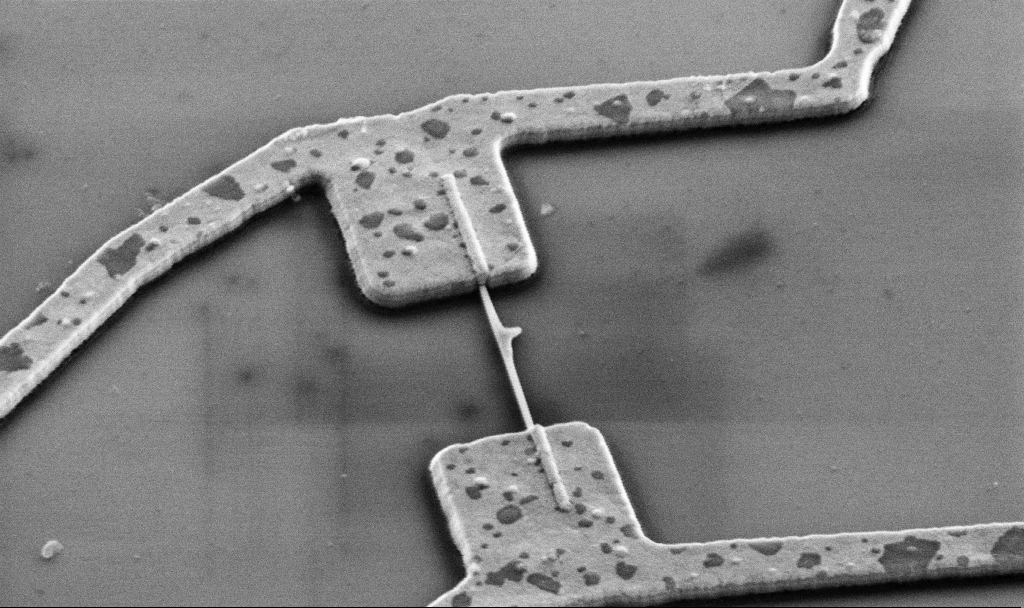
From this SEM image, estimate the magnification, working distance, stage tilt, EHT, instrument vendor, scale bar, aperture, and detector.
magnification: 30 K X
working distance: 15.7 mm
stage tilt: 45°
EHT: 5 kV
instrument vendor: Zeiss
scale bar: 1000 nm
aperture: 30 µm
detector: SE2